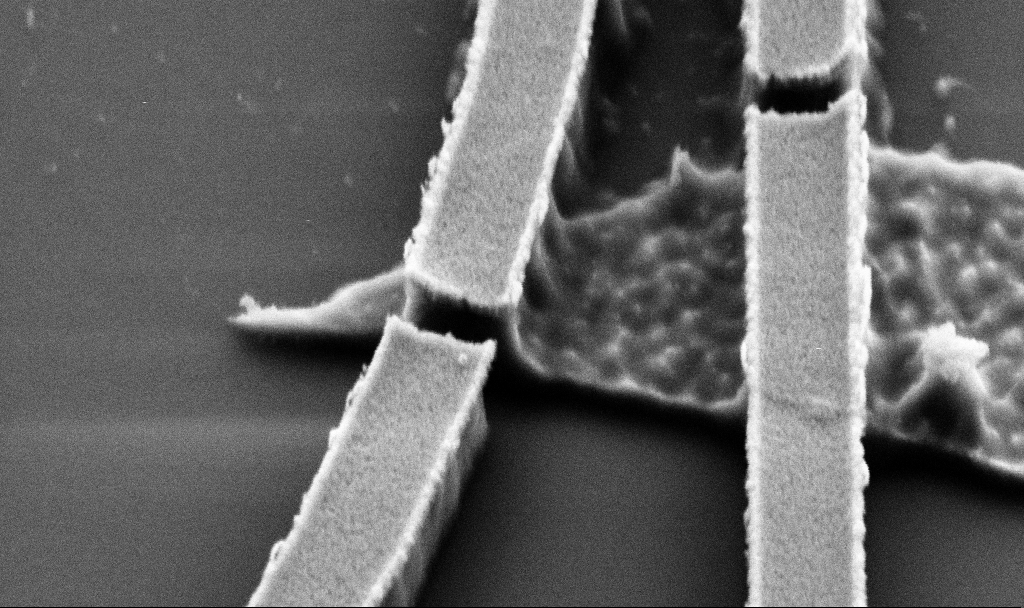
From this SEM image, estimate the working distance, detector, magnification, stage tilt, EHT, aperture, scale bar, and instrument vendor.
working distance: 7.2 mm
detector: SE2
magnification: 85.84 K X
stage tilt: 45°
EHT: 3 kV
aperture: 30 µm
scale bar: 200 nm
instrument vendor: Zeiss